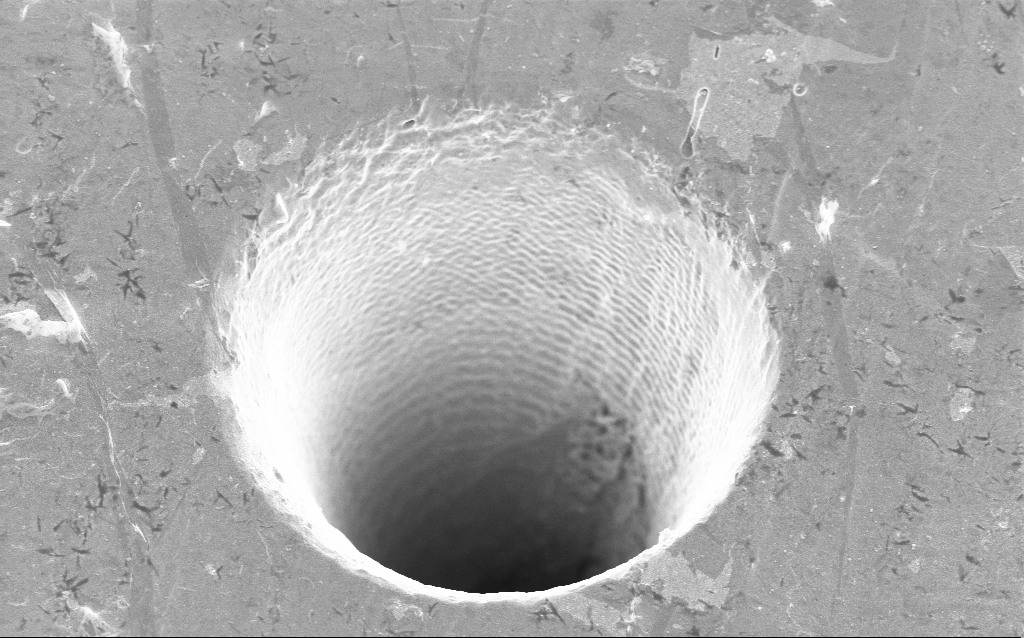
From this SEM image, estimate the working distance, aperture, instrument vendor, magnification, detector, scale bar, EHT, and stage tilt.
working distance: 4.3 mm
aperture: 30 µm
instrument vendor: Zeiss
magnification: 13.46 K X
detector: InLens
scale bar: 2000 nm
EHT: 5 kV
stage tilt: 19.6°